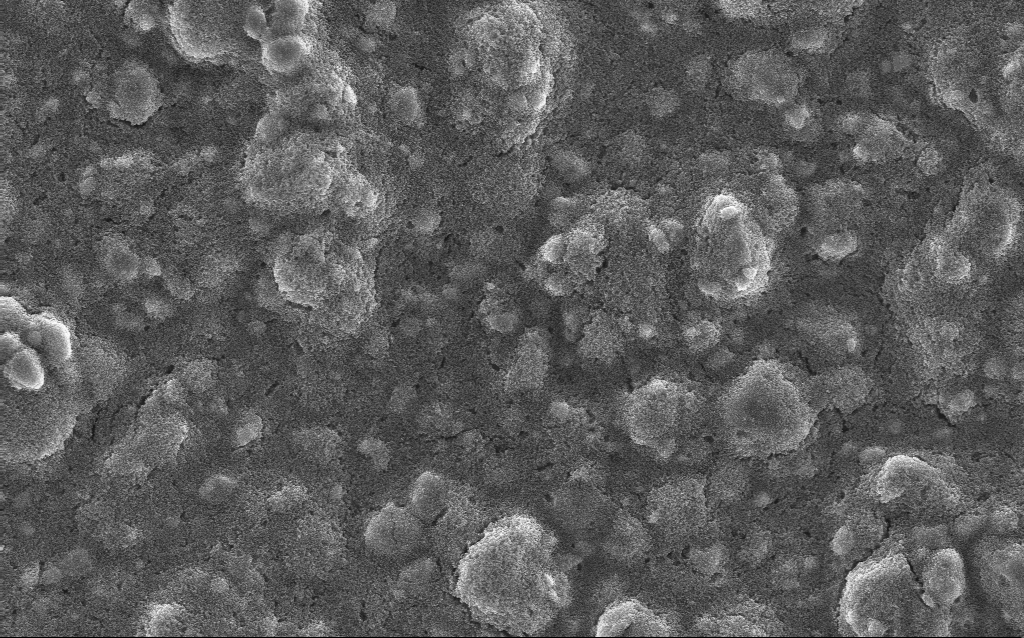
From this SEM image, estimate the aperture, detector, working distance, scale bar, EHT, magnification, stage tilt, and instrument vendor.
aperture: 30 µm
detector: InLens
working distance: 2.8 mm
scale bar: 10000 nm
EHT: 10 kV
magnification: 5 K X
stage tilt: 0°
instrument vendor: Zeiss